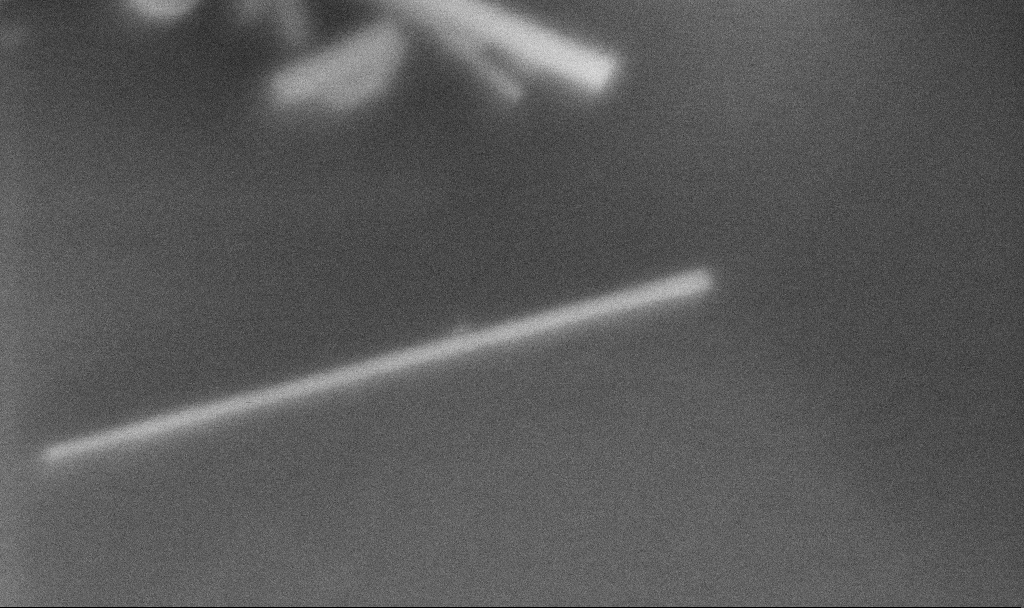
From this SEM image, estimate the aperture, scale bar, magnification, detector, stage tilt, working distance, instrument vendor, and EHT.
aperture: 30 µm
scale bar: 100 nm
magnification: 414.43 K X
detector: InLens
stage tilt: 0°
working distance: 3.3 mm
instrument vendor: Zeiss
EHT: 3 kV